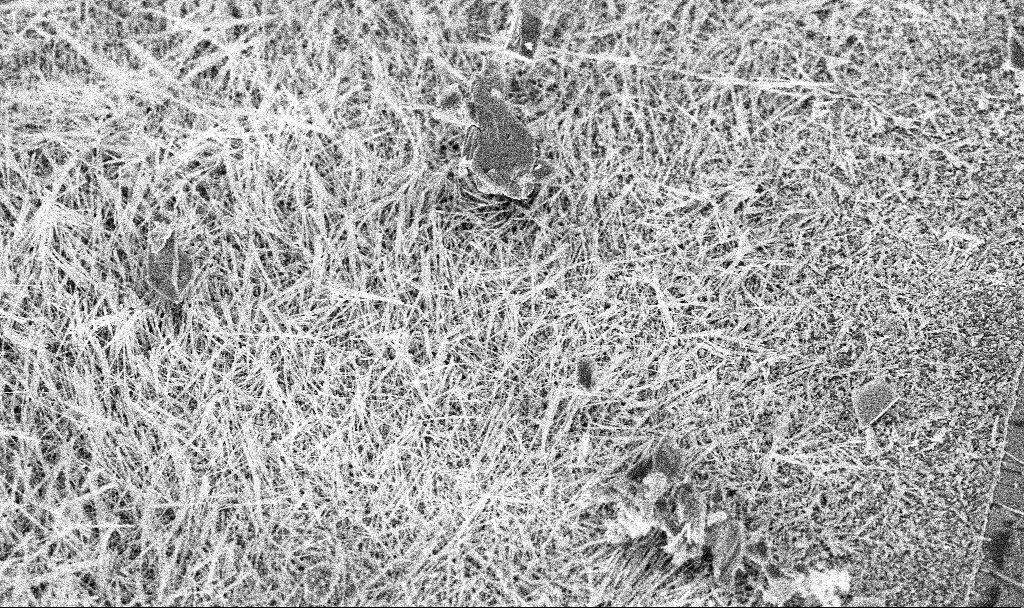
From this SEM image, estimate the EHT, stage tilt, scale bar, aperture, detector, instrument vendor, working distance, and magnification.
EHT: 10 kV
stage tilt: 45°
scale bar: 10000 nm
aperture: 30 µm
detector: InLens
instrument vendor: Zeiss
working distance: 7 mm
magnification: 4.48 K X